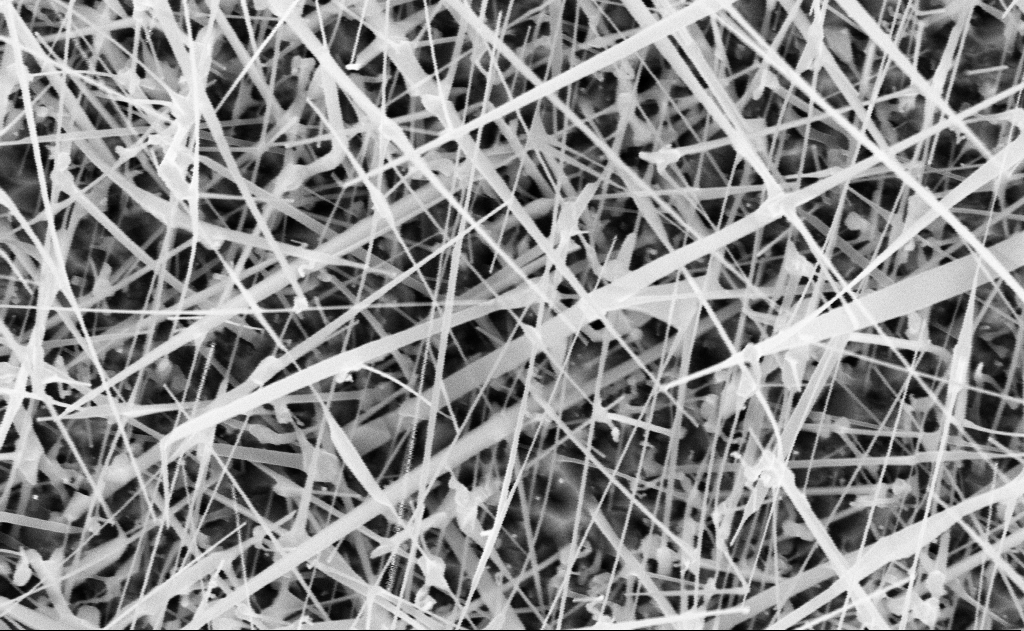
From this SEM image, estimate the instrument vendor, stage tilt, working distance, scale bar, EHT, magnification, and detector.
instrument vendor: Zeiss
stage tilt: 0°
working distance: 20 mm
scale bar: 1000 nm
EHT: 10 kV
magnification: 40 K X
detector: InLens